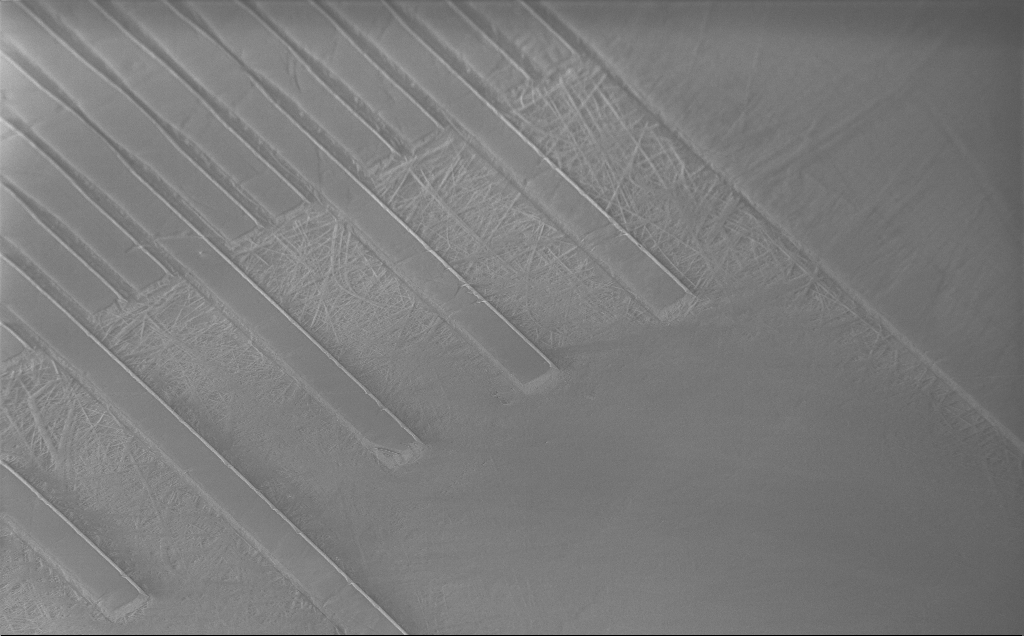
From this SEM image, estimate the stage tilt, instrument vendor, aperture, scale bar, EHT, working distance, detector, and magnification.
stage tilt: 45°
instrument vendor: Zeiss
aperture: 30 µm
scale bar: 100000 nm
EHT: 3 kV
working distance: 9 mm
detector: InLens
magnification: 0.194 K X